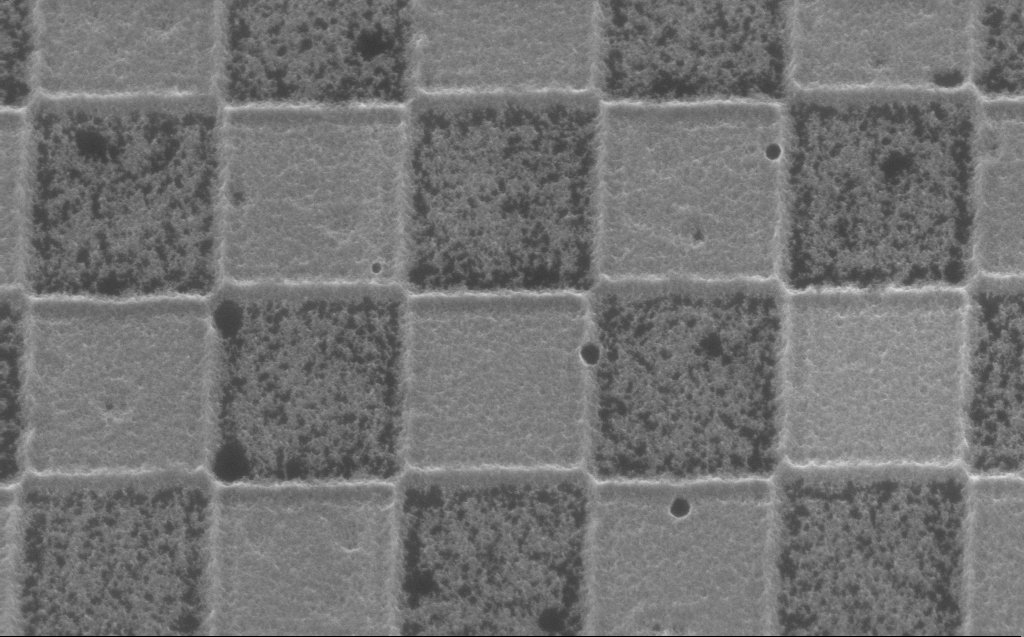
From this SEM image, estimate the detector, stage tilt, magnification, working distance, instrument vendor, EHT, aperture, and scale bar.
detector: InLens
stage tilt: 30°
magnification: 70.38 K X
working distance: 4 mm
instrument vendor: Zeiss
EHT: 5 kV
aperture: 30 µm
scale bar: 1000 nm